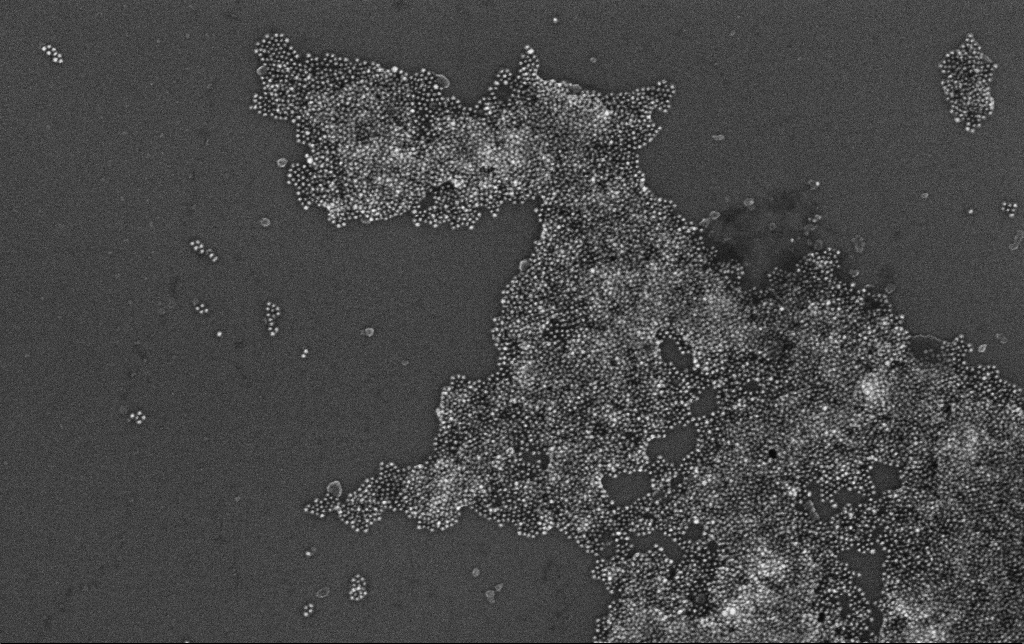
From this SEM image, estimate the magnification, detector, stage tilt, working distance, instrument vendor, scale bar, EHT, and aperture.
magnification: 100 K X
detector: InLens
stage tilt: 0°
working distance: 3.4 mm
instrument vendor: Zeiss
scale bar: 200 nm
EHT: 10 kV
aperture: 30 µm